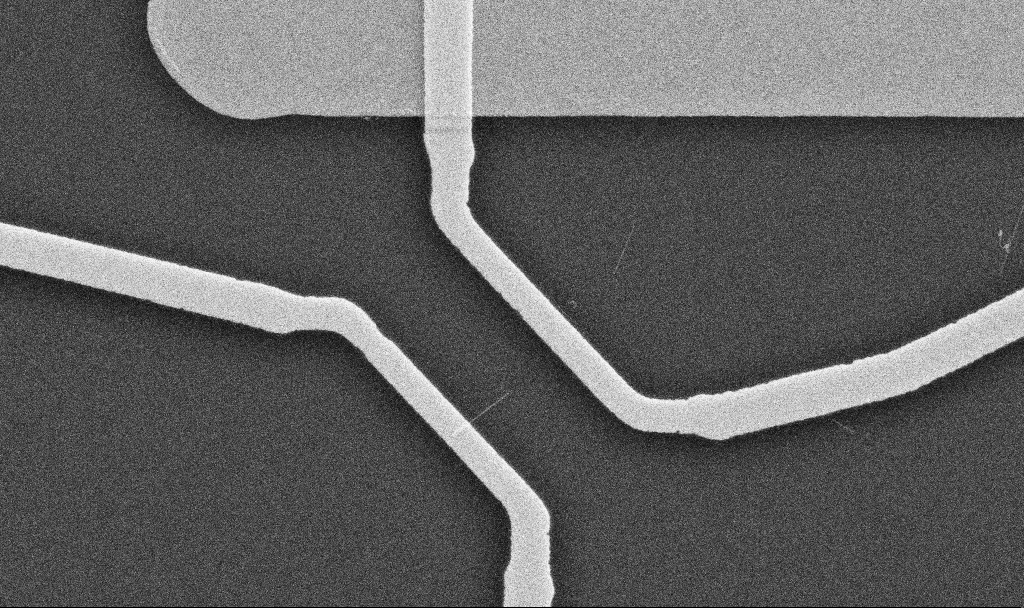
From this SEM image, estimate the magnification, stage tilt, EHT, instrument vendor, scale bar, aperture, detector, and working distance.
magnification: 20 K X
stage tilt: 0°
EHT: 10 kV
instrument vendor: Zeiss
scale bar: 1000 nm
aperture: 30 µm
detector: SE2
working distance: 10.7 mm